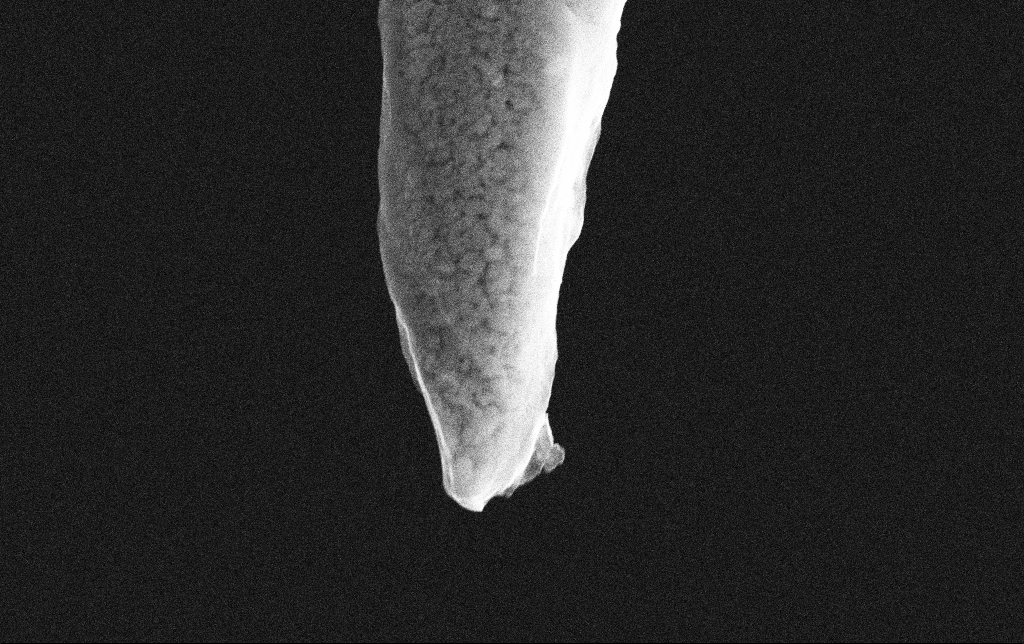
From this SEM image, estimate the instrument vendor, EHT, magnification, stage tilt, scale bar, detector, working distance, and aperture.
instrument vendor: Zeiss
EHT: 10 kV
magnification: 56.97 K X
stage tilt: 0°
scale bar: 1000 nm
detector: SE2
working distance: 8.7 mm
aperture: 30 µm